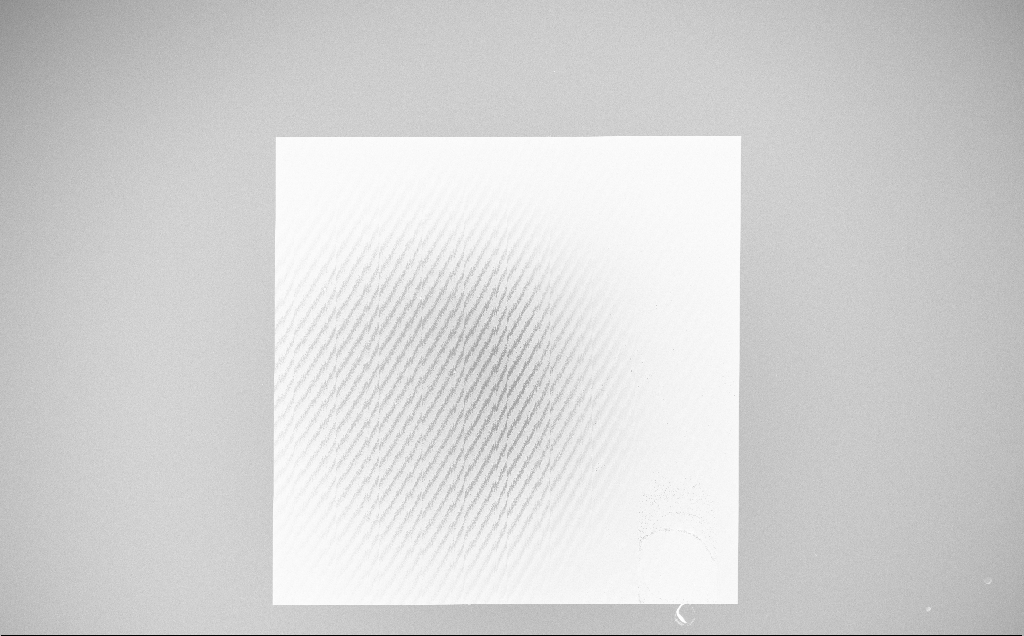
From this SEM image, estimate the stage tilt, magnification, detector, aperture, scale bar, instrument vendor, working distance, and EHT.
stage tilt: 0°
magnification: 0.174 K X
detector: InLens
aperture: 30 µm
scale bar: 100000 nm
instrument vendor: Zeiss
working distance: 6 mm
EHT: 10 kV